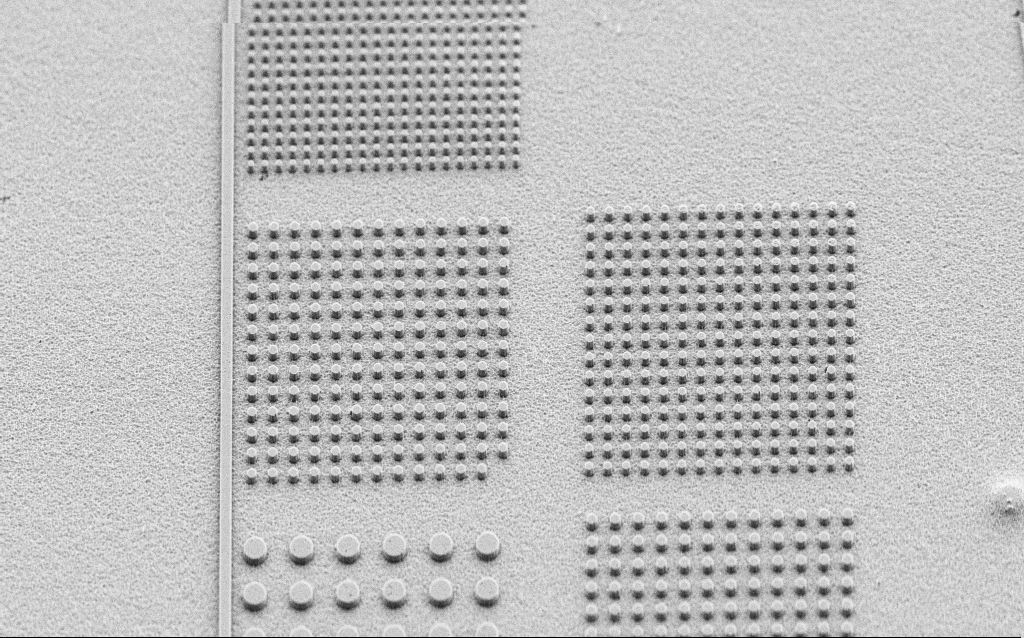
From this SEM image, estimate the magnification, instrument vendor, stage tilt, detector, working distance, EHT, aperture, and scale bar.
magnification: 4.33 K X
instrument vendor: Zeiss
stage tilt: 45°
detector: SE2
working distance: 5 mm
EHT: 3 kV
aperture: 30 µm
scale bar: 10000 nm